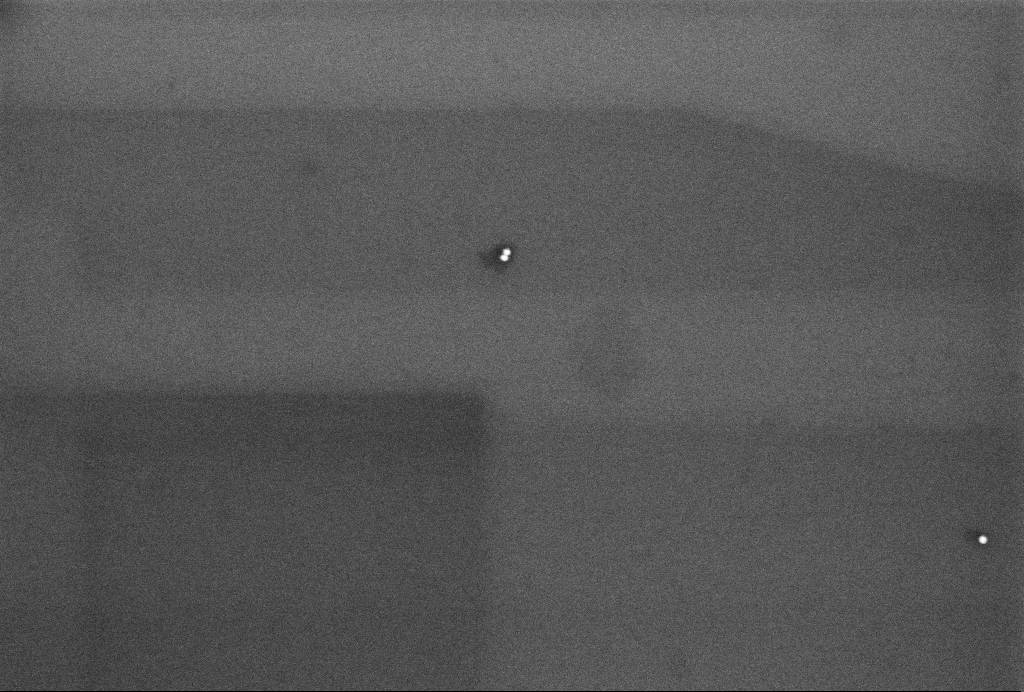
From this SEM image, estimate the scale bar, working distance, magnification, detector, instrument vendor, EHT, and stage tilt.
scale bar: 200 nm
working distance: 3.2 mm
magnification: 104.07 K X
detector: InLens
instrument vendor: Zeiss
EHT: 3 kV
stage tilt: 0°